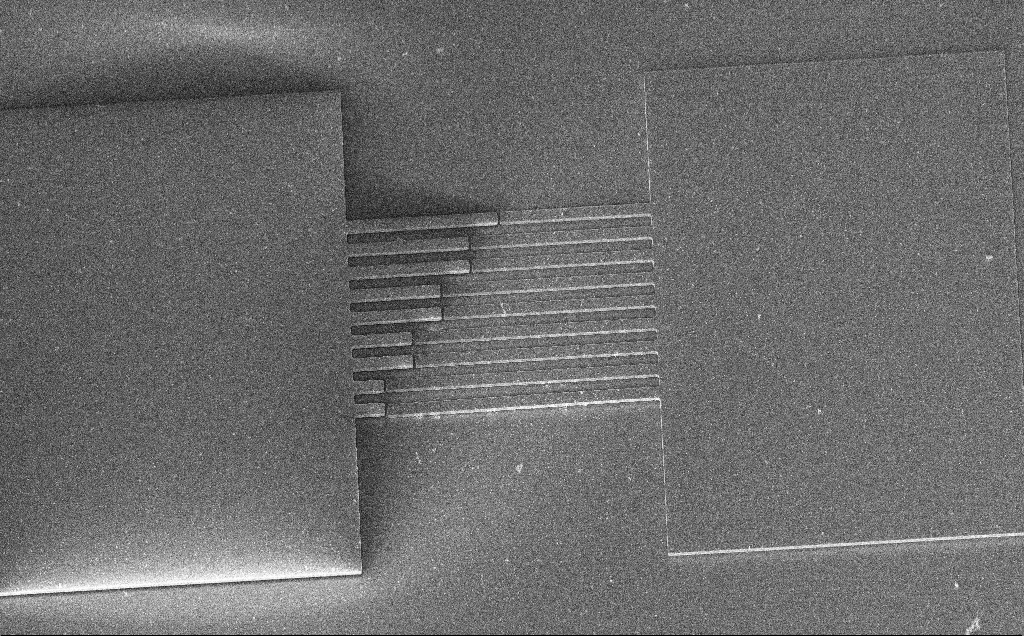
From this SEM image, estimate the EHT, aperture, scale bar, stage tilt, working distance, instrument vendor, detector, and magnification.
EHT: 7.5 kV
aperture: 30 µm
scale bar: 100000 nm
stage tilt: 45°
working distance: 10 mm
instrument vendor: Zeiss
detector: InLens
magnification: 0.448 K X